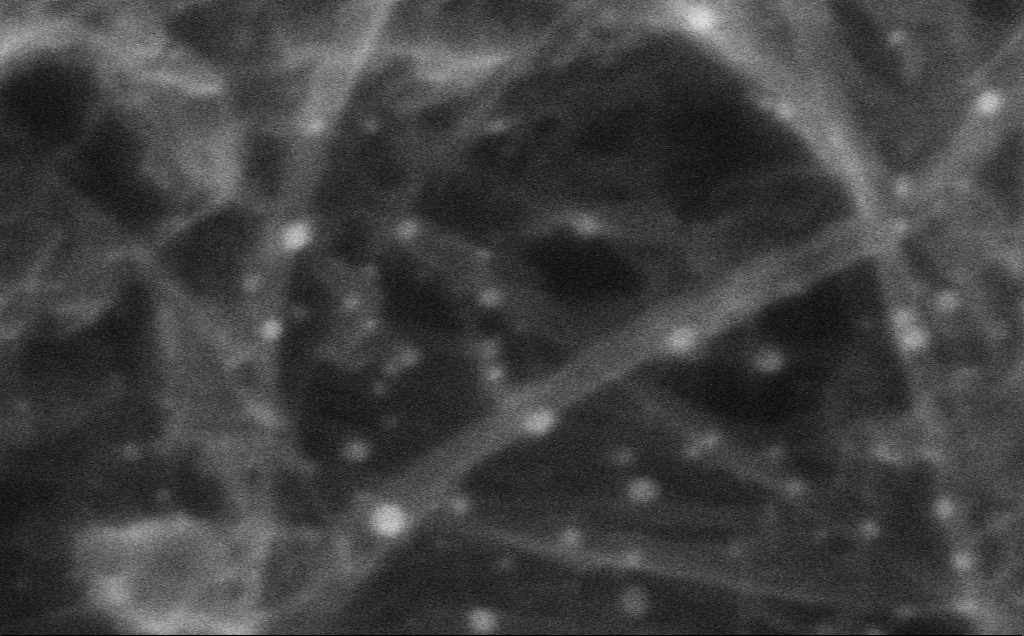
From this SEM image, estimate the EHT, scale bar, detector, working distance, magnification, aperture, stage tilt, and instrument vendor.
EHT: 10 kV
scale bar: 20 nm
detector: InLens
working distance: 3 mm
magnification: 978.84 K X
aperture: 30 µm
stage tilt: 0°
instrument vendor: Zeiss